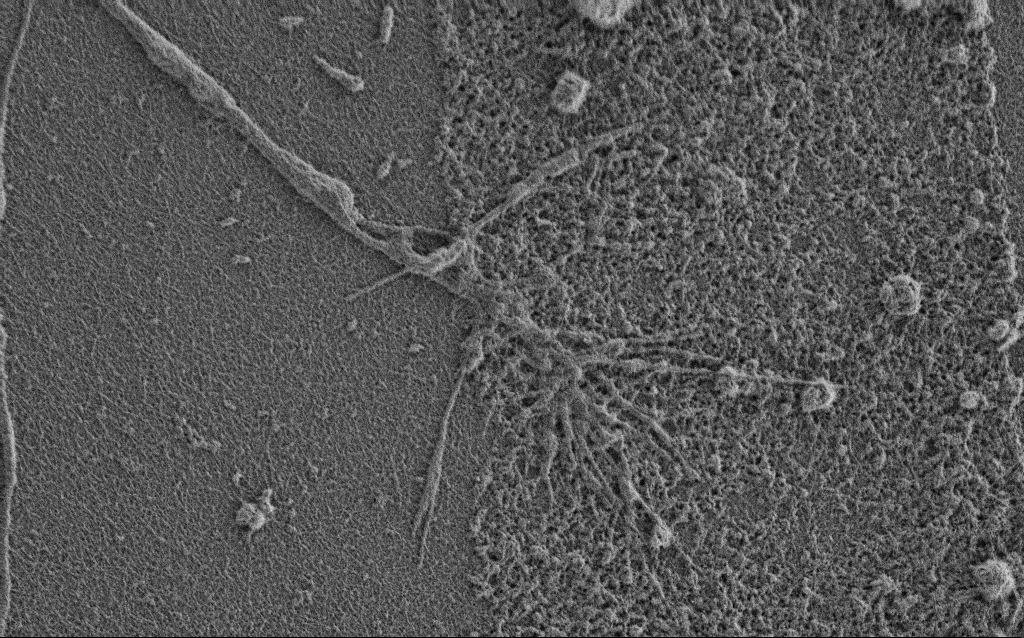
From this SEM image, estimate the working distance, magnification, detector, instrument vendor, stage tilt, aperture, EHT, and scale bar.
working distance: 3.4 mm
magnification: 10 K X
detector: SE2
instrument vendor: Zeiss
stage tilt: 0°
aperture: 30 µm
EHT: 0.9 kV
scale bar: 2000 nm